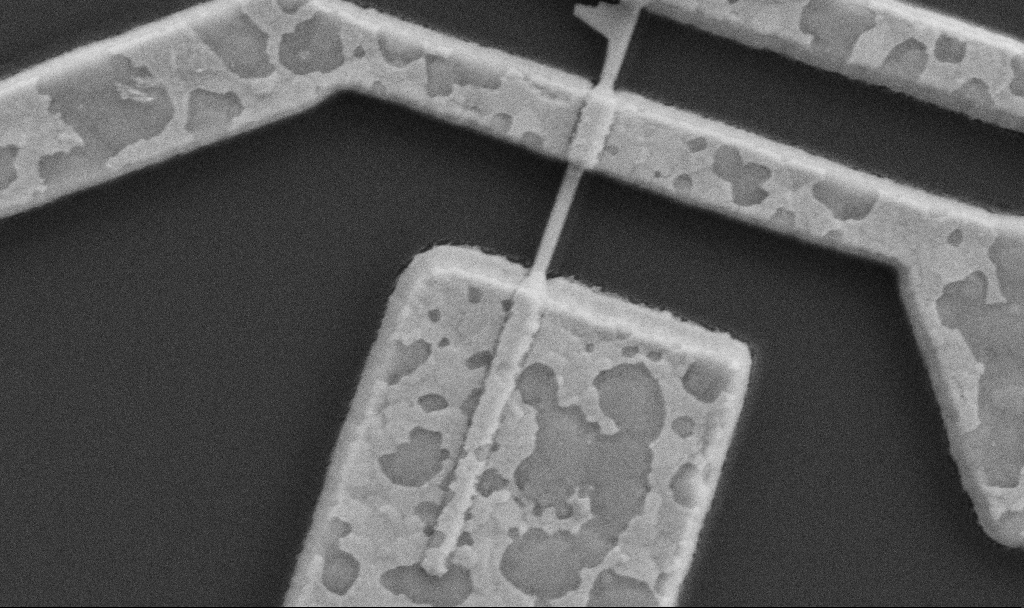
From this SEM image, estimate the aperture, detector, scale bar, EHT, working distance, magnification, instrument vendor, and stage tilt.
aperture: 30 µm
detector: SE2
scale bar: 1000 nm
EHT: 5 kV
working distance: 8.7 mm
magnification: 60 K X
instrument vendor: Zeiss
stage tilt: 0°